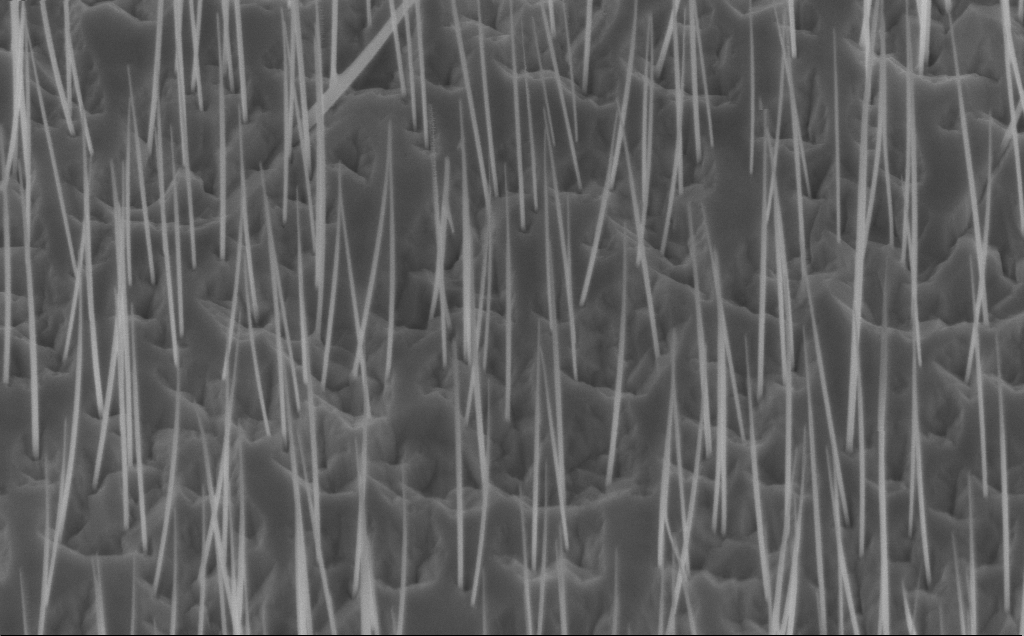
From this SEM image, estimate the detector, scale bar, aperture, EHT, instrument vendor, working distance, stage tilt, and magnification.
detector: InLens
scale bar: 1000 nm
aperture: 30 µm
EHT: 5 kV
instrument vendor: Zeiss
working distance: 6 mm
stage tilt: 45°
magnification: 40 K X